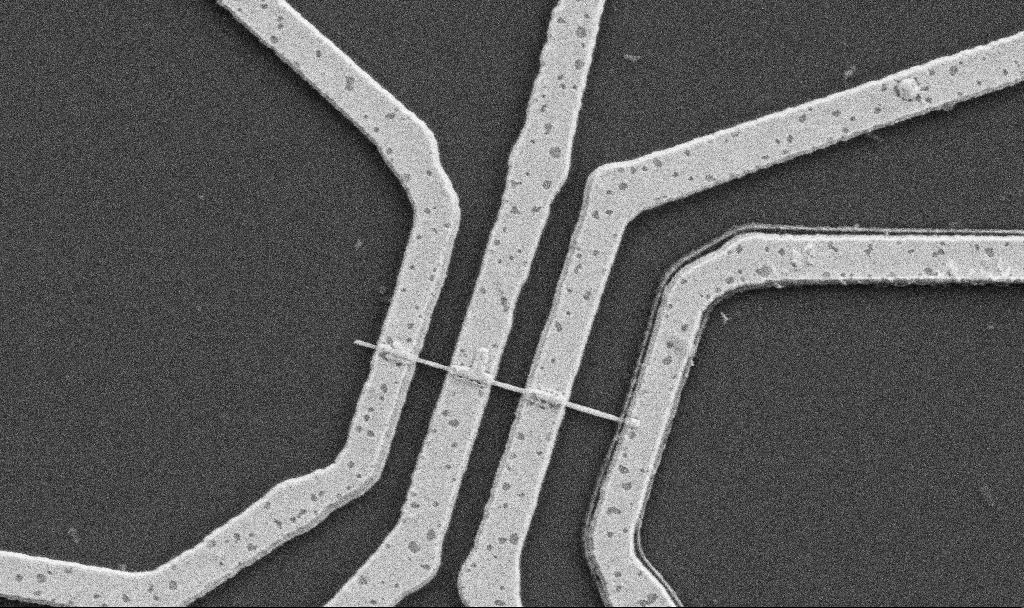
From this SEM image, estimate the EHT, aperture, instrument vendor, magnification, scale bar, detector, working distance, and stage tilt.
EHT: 5 kV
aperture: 30 µm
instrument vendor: Zeiss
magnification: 20 K X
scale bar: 1000 nm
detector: SE2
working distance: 10.7 mm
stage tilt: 0°